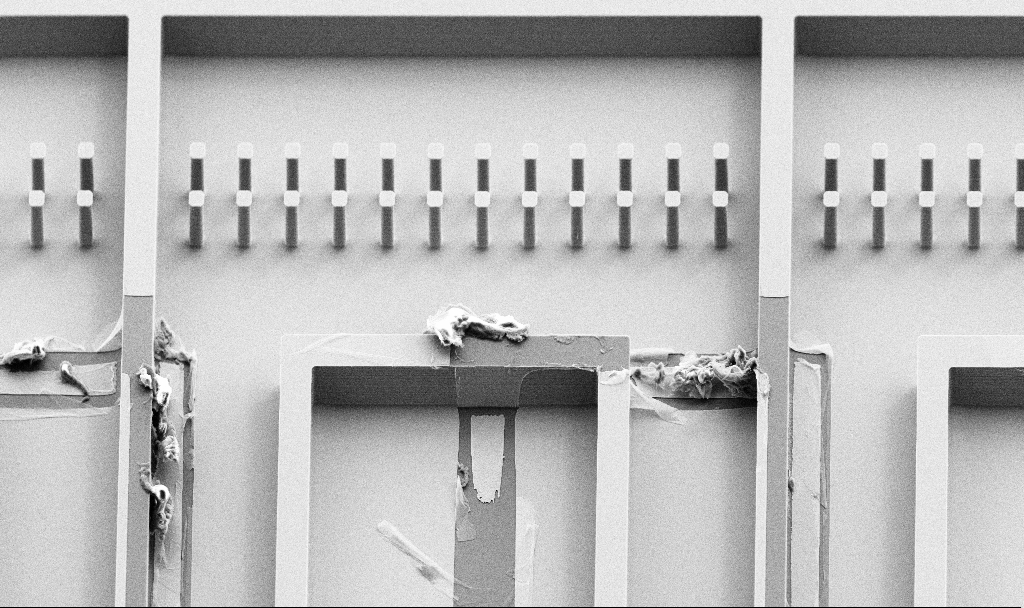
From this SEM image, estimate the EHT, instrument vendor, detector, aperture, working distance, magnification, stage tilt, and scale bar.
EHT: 10 kV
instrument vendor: Zeiss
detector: SE2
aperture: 30 µm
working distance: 6.6 mm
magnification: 0.588 K X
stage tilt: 45°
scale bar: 100000 nm